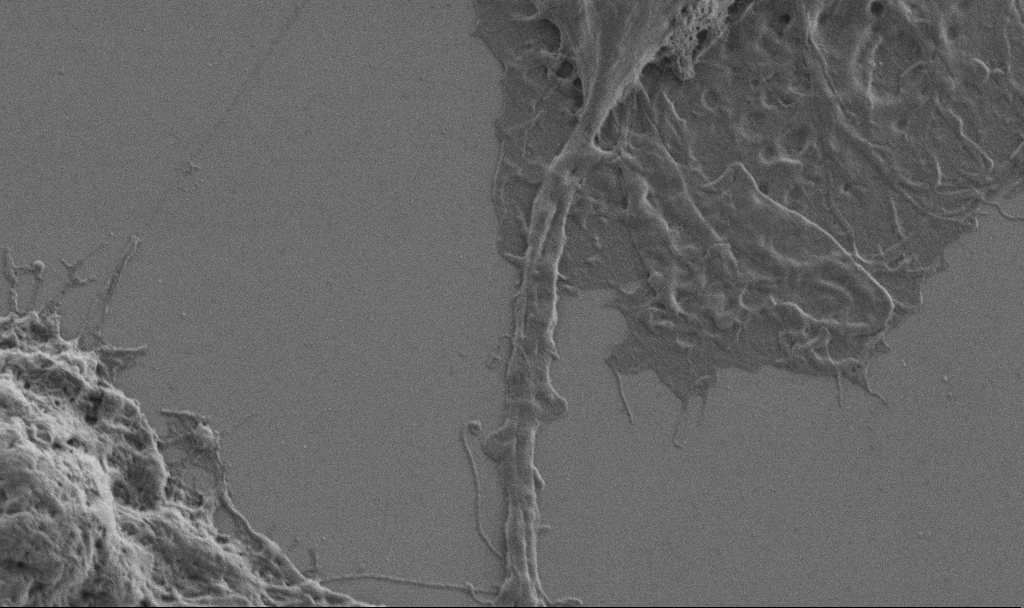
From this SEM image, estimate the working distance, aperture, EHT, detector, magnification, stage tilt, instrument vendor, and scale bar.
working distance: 6.9 mm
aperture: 30 µm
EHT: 1 kV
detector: SE2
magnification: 10 K X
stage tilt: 0°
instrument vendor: Zeiss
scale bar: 2000 nm